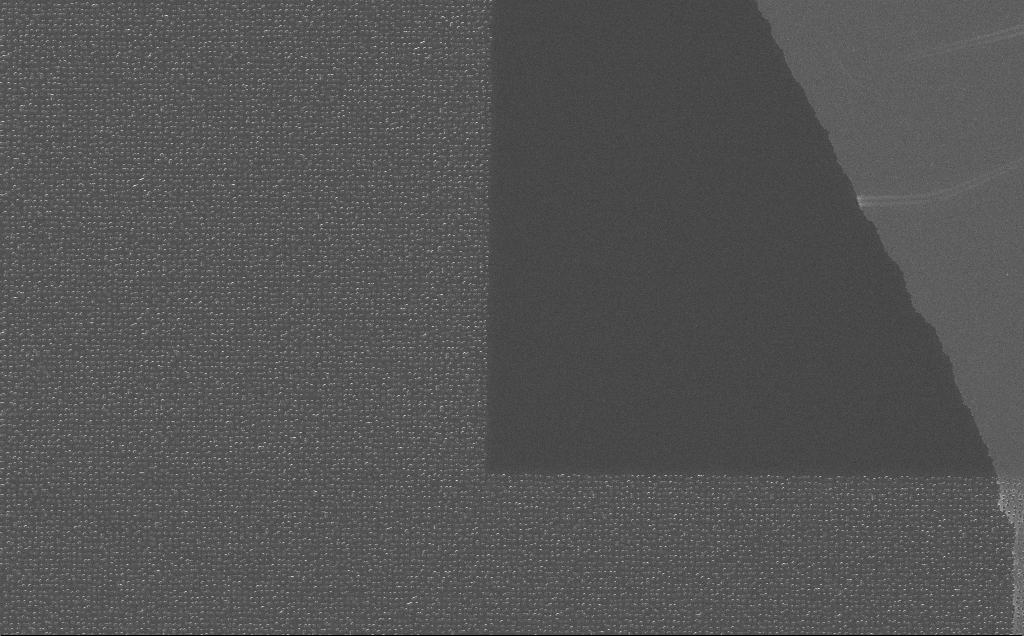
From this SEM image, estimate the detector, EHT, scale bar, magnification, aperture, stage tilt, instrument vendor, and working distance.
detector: InLens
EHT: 10 kV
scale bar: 10000 nm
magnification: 4.95 K X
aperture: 30 µm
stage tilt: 0°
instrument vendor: Zeiss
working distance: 7 mm